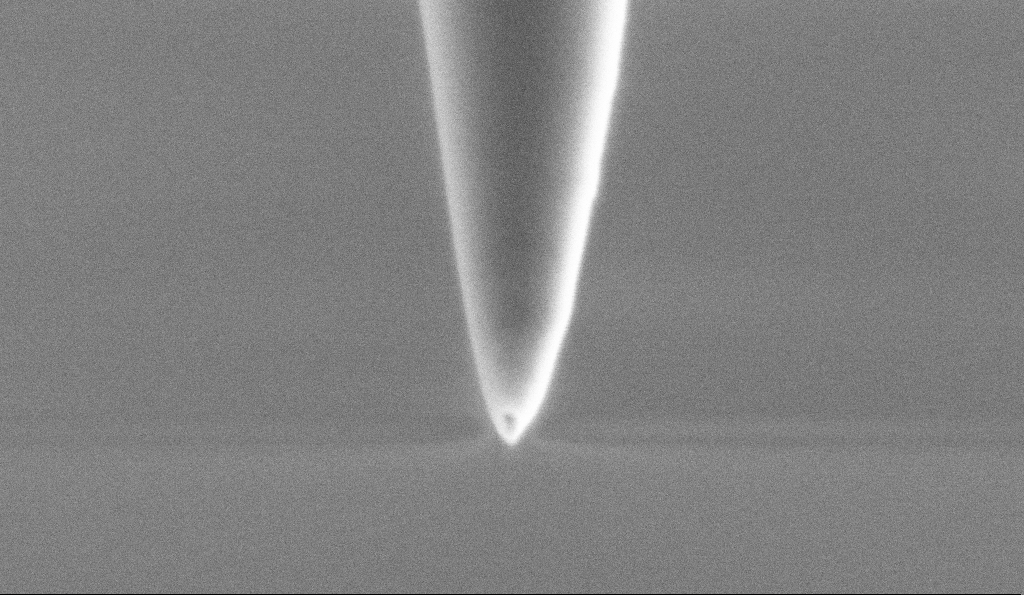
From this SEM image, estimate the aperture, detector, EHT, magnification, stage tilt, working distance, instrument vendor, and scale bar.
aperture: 30 µm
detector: SE2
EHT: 1 kV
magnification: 150 K X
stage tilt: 45°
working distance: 6.6 mm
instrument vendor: Zeiss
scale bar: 200 nm